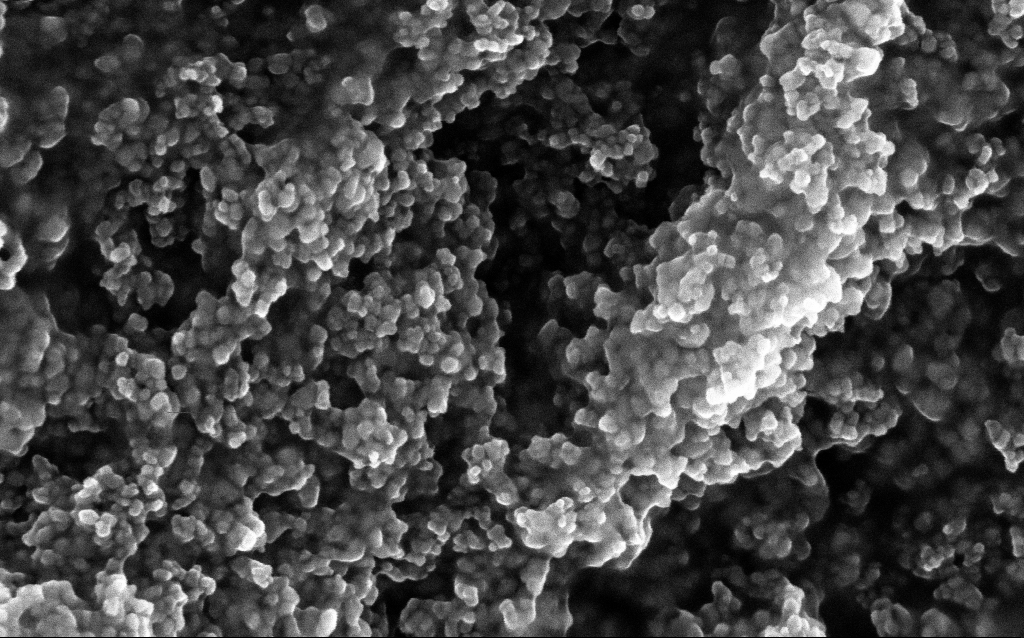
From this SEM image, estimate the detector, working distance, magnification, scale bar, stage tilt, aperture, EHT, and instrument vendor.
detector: InLens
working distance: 2.6 mm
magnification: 211.33 K X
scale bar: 100 nm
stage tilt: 0°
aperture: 30 µm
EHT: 10 kV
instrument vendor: Zeiss